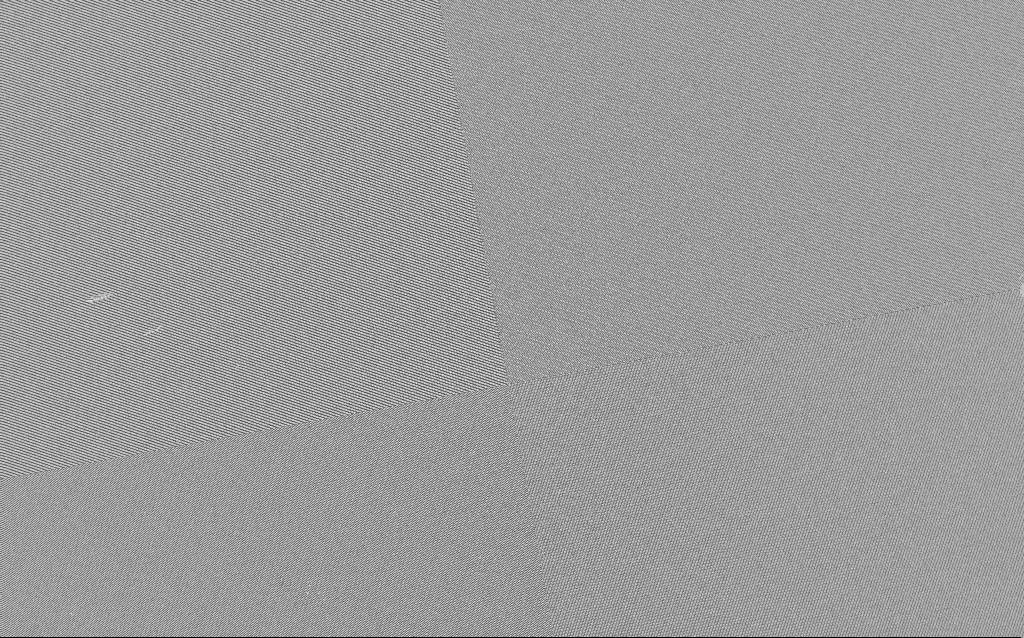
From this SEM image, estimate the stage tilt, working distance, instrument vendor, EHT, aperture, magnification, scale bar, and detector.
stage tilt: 0°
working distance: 4.5 mm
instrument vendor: Zeiss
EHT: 3 kV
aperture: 30 µm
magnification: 1.41 K X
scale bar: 10000 nm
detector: SE2